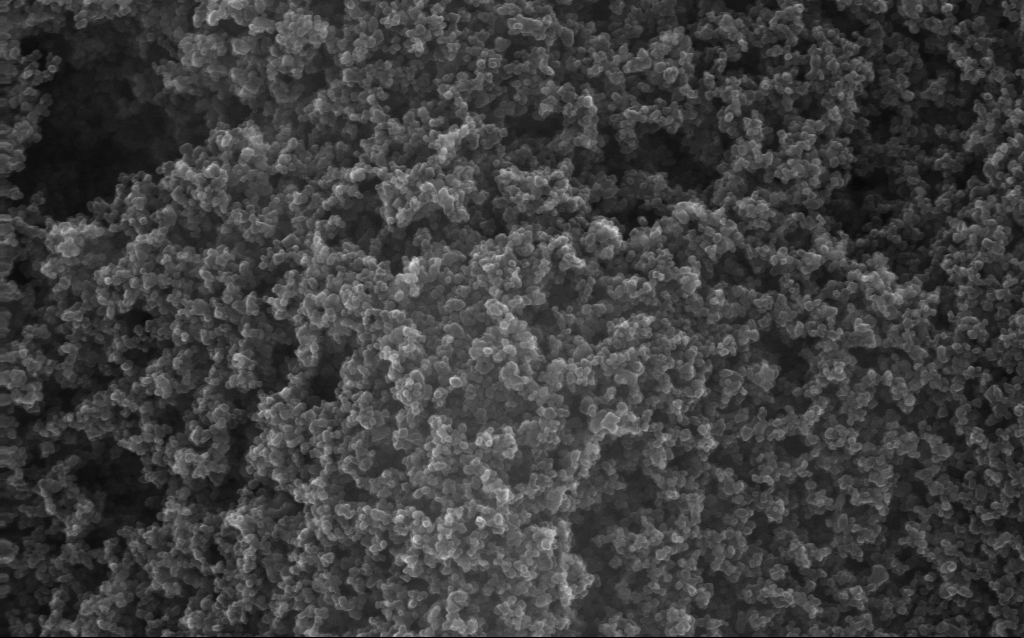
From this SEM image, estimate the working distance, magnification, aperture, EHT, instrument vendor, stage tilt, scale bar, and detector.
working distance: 3 mm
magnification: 130 K X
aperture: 30 µm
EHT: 20 kV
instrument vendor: Zeiss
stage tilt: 0°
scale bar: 100 nm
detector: InLens